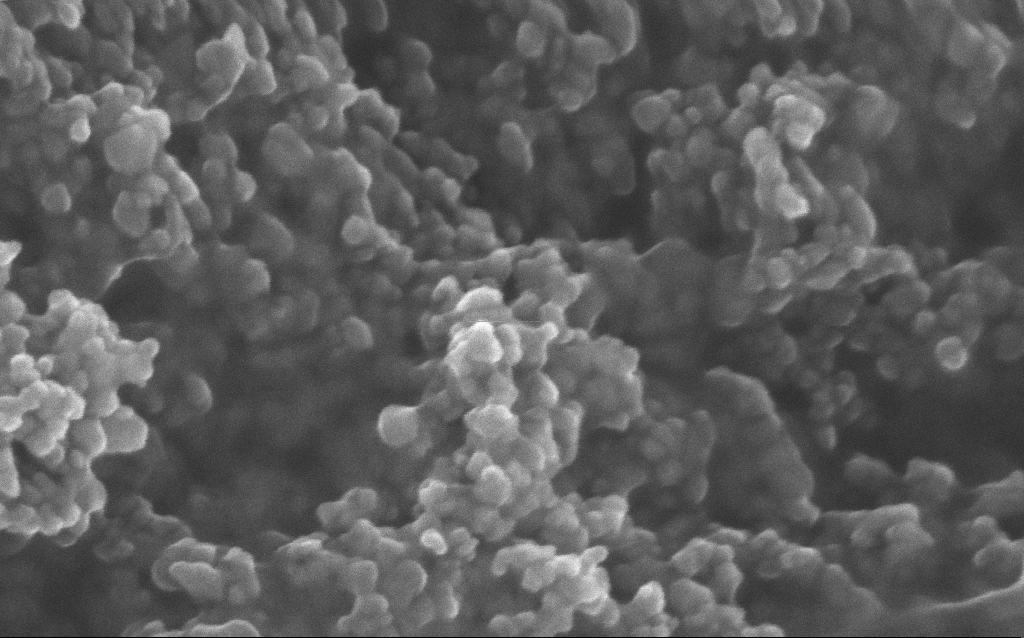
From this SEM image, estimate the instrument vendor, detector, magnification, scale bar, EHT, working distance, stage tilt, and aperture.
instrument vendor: Zeiss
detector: InLens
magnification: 348.1 K X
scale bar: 100 nm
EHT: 10 kV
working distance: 2.8 mm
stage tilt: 0°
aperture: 30 µm